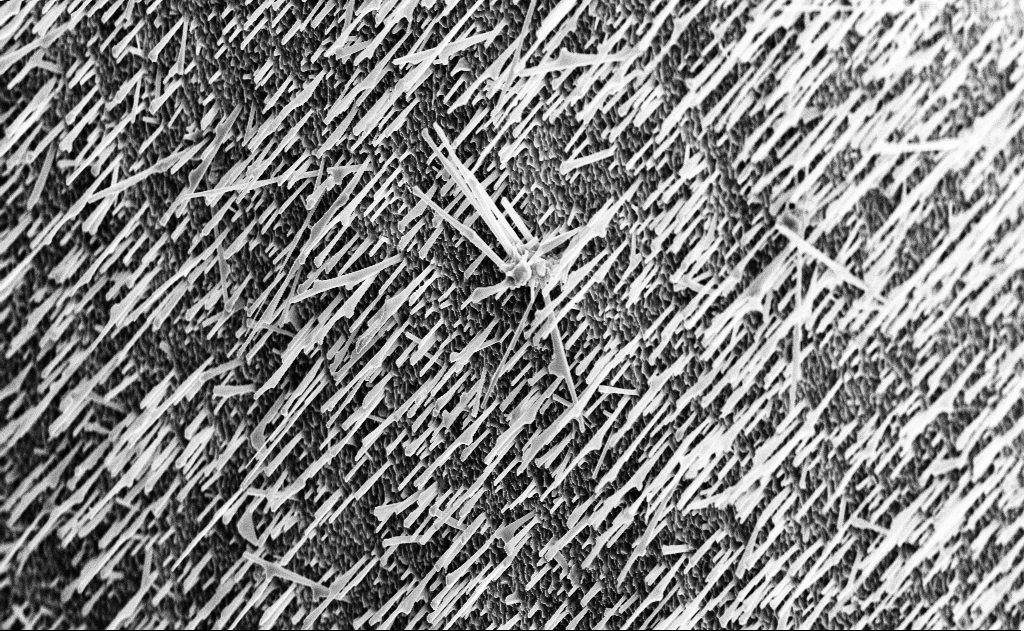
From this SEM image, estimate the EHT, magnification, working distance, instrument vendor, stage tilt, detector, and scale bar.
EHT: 10 kV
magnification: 5 K X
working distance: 15 mm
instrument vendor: Zeiss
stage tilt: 0°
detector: InLens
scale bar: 10000 nm